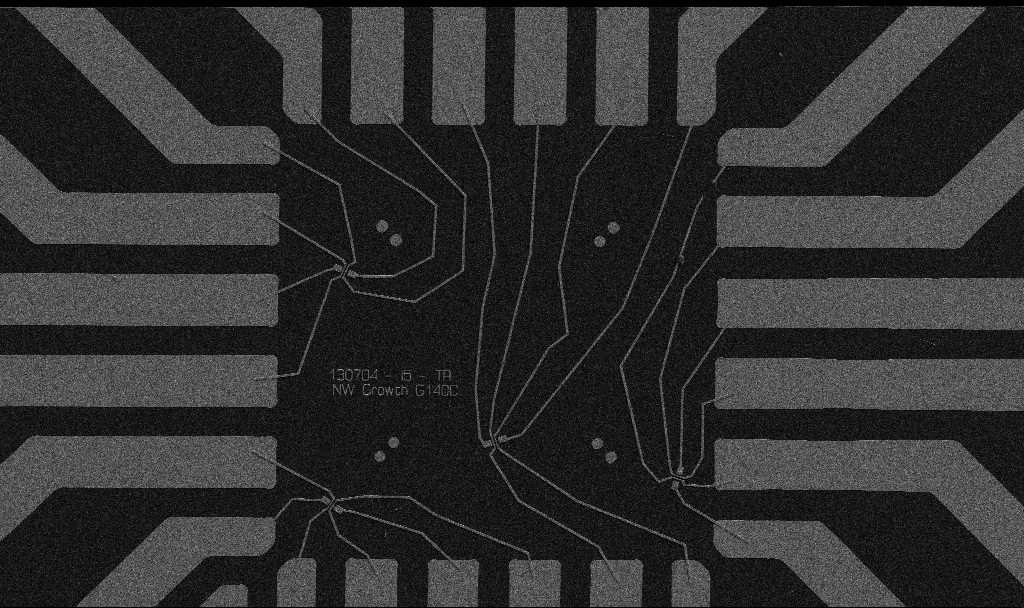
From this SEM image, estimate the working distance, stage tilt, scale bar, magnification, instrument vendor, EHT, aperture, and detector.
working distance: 10.7 mm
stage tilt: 0°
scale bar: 20000 nm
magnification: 1 K X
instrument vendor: Zeiss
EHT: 5 kV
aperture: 30 µm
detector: SE2